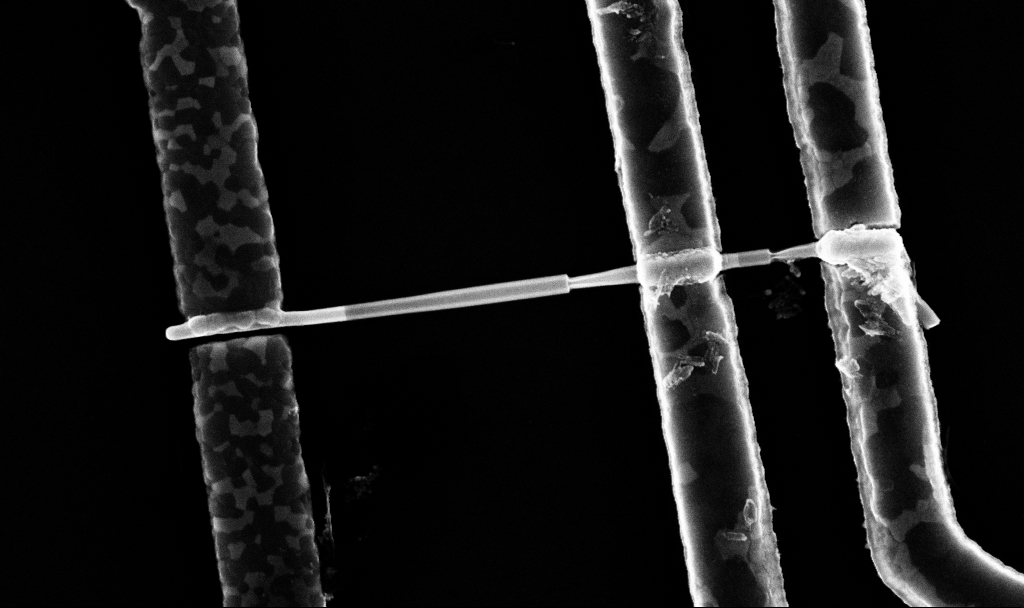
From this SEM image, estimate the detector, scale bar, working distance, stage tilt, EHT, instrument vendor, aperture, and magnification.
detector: InLens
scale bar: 1000 nm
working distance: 7.7 mm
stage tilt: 0°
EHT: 10 kV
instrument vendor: Zeiss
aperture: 30 µm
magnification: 53.26 K X